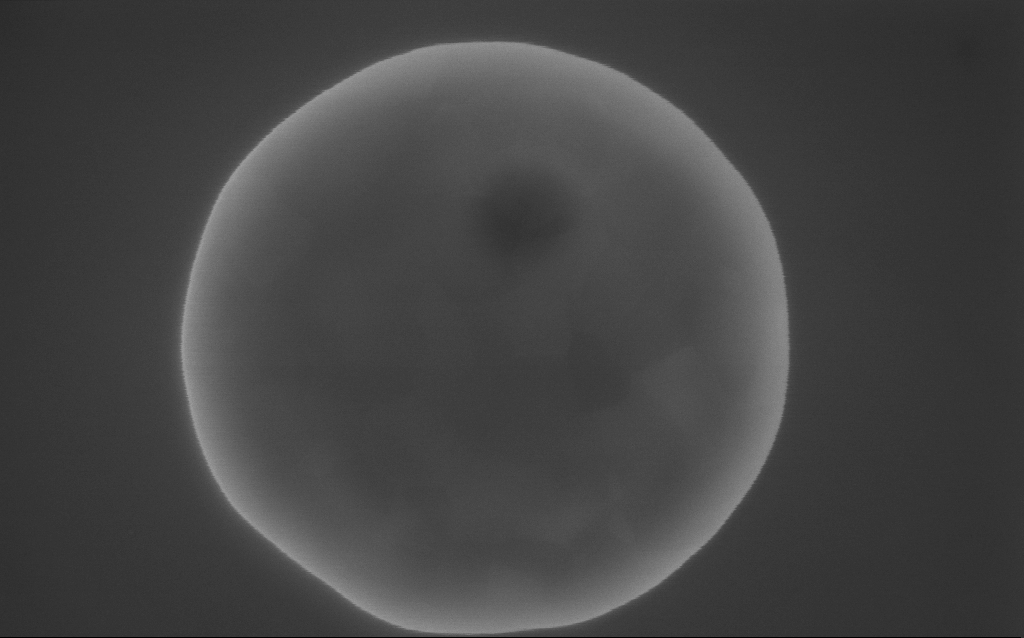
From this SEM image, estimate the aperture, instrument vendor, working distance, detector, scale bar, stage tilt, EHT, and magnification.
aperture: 30 µm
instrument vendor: Zeiss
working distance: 3 mm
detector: InLens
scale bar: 200 nm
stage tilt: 0°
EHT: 10 kV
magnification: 175 K X